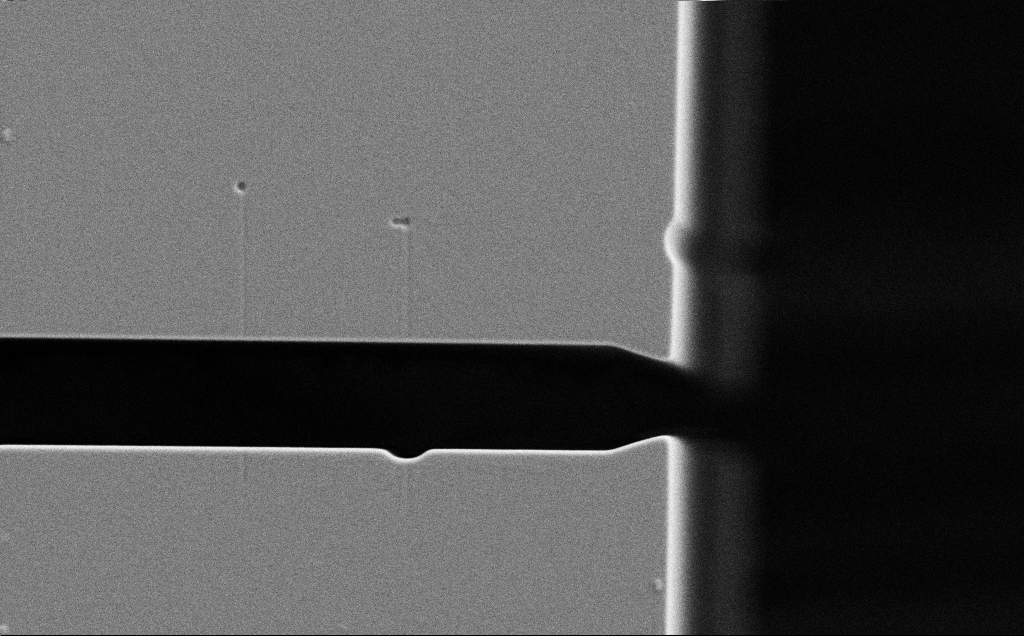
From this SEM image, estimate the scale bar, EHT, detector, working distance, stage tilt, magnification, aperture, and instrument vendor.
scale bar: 20000 nm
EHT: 5 kV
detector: SE2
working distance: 7 mm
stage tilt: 0°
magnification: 3.3 K X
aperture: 30 µm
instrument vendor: Zeiss